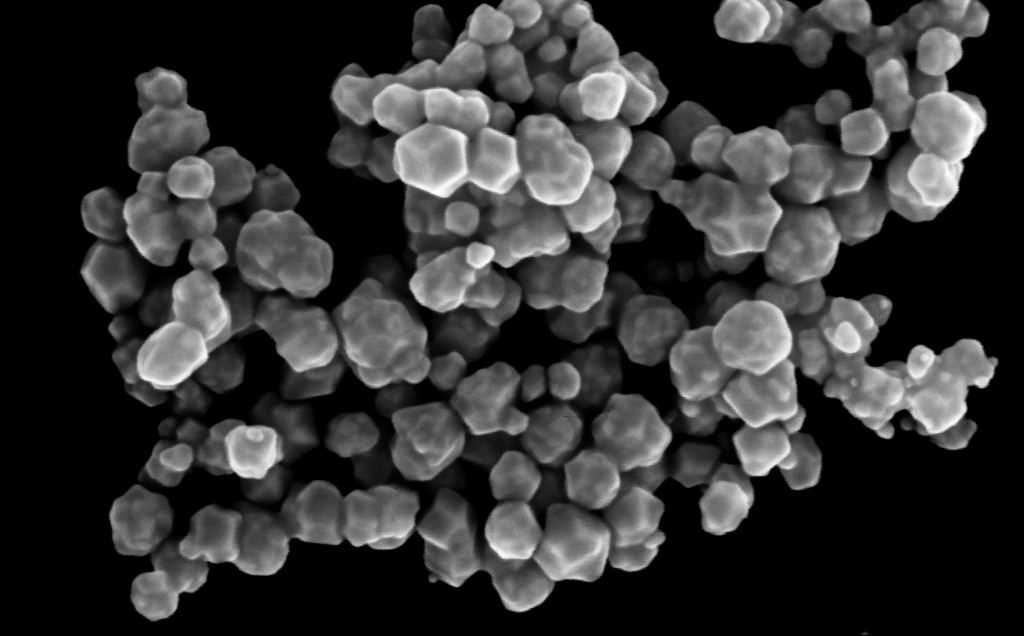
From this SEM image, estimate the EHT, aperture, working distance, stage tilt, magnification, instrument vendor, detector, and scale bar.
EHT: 10 kV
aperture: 30 µm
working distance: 3 mm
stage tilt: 0°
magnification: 296.05 K X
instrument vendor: Zeiss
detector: InLens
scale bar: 200 nm